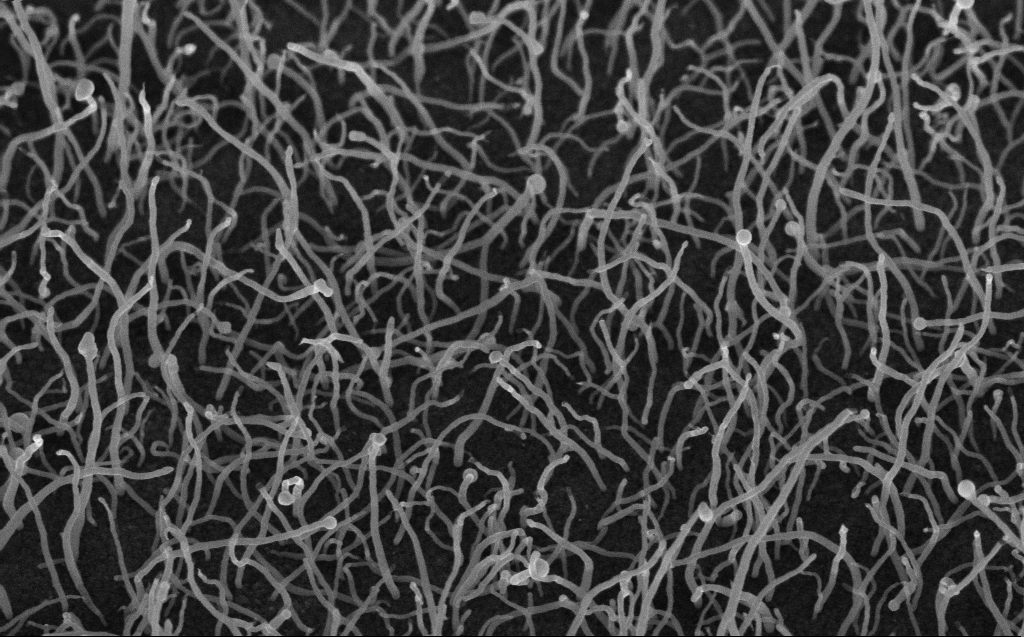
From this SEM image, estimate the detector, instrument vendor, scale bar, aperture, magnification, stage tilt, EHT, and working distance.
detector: InLens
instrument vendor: Zeiss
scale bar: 1000 nm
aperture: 30 µm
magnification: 50 K X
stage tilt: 45°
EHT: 10 kV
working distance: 6 mm